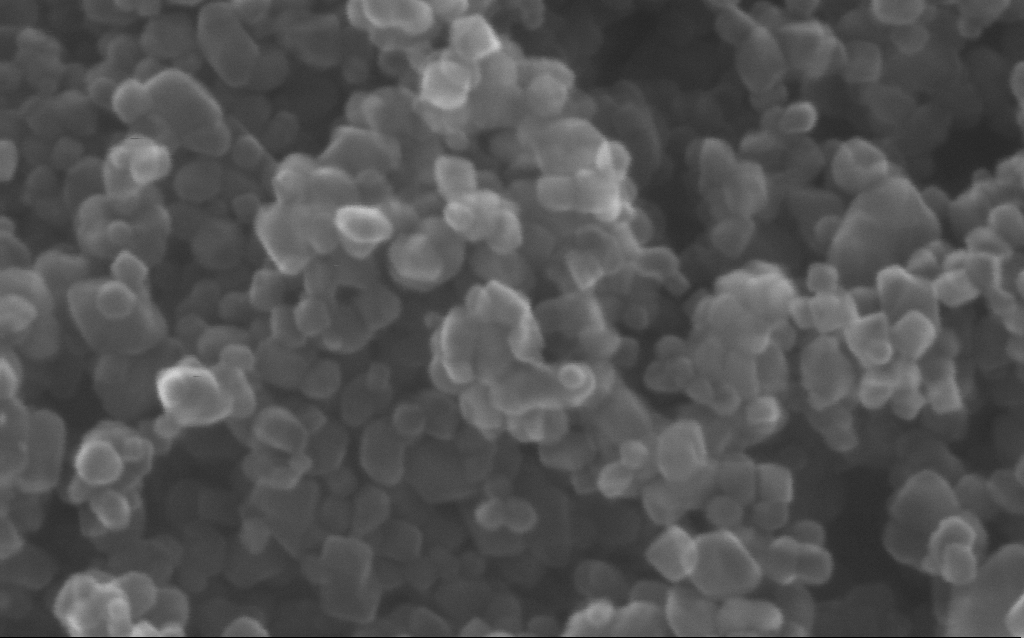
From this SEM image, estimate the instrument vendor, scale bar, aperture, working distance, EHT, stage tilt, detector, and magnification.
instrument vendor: Zeiss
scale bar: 100 nm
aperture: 30 µm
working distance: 3 mm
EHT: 20 kV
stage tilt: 0°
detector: InLens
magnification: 716 K X